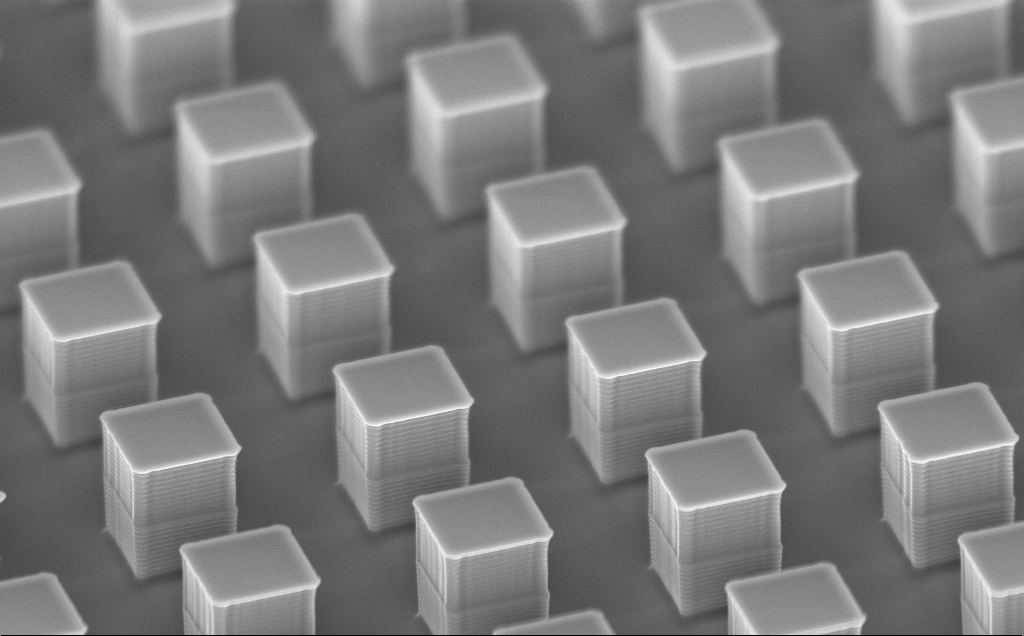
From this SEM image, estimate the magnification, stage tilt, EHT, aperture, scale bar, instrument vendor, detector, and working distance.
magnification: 4.5 K X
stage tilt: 59.6°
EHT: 10 kV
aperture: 120 µm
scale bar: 10000 nm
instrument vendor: Zeiss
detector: InLens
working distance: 15 mm